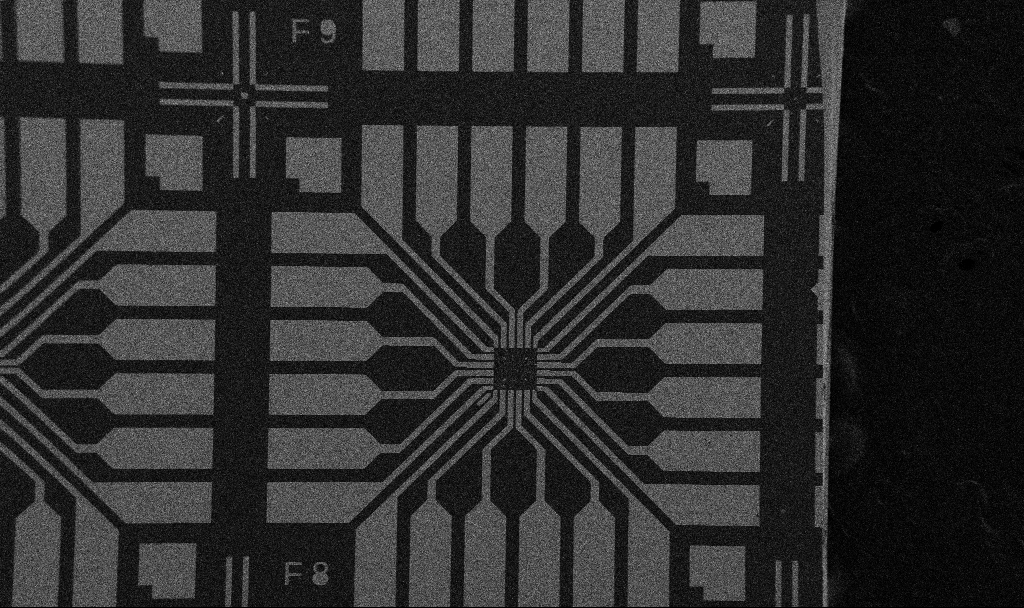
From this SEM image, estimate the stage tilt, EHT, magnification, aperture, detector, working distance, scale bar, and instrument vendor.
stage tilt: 0°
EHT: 5 kV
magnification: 0.1 K X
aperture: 30 µm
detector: SE2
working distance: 10.7 mm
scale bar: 200000 nm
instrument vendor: Zeiss